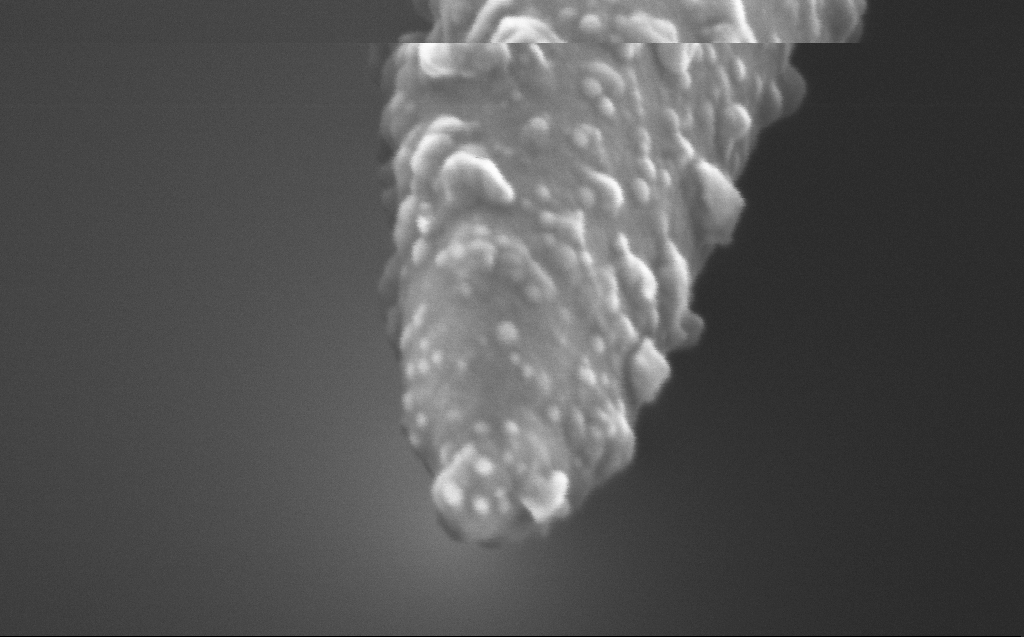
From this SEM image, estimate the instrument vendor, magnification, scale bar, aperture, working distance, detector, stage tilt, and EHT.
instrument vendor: Zeiss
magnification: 500 K X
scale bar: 100 nm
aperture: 30 µm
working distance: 3 mm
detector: InLens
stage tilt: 45°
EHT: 5 kV